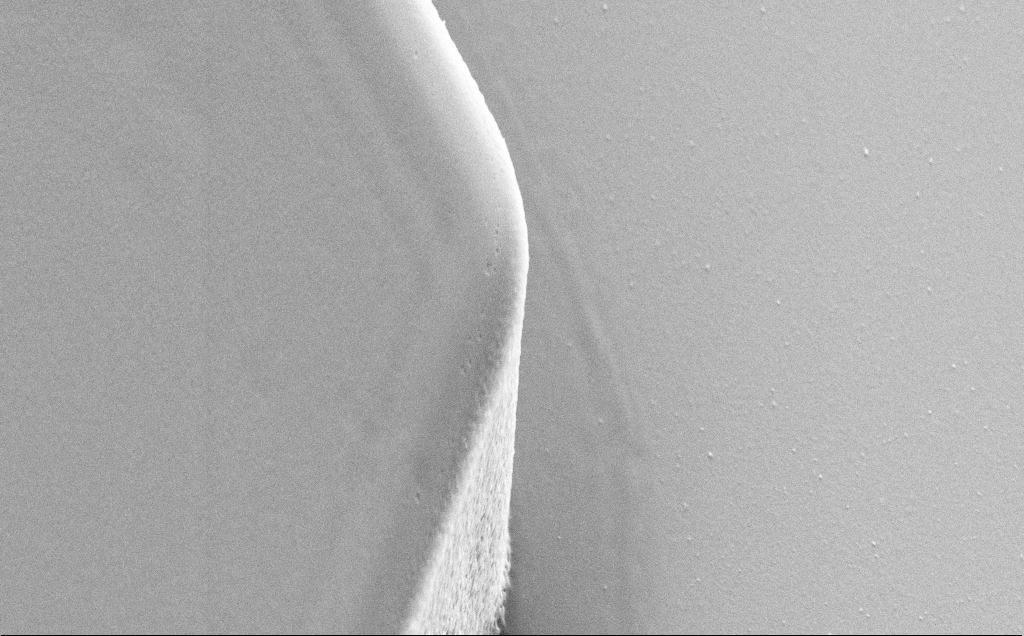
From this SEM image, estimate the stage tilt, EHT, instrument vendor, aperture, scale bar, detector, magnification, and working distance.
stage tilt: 30°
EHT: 5 kV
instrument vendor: Zeiss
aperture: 30 µm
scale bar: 2000 nm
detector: SE2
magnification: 12.57 K X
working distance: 9 mm